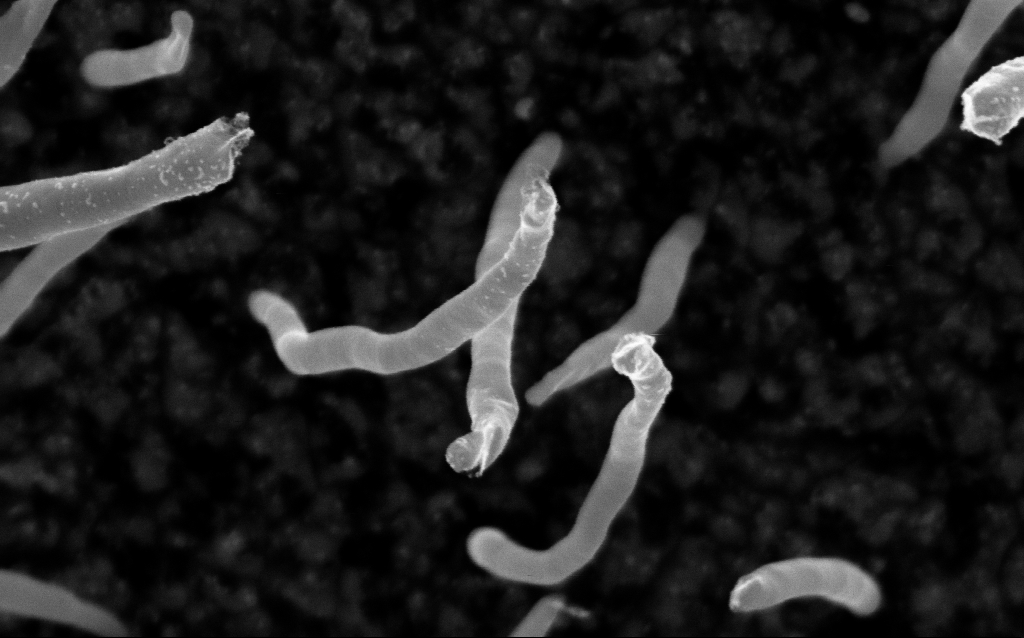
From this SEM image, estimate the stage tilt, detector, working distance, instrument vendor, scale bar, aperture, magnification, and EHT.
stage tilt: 0°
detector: InLens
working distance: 1.5 mm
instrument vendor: Zeiss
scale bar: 100 nm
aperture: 30 µm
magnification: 200 K X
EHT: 5 kV